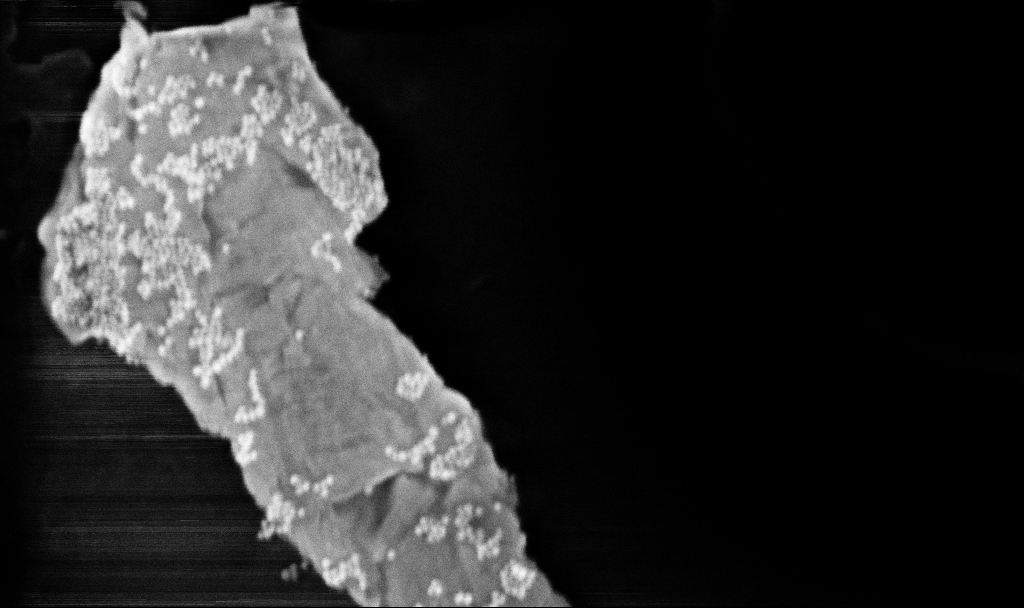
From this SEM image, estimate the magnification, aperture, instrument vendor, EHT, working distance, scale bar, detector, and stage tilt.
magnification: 158.01 K X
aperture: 30 µm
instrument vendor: Zeiss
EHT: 5 kV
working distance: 3.7 mm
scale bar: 200 nm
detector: InLens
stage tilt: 0°